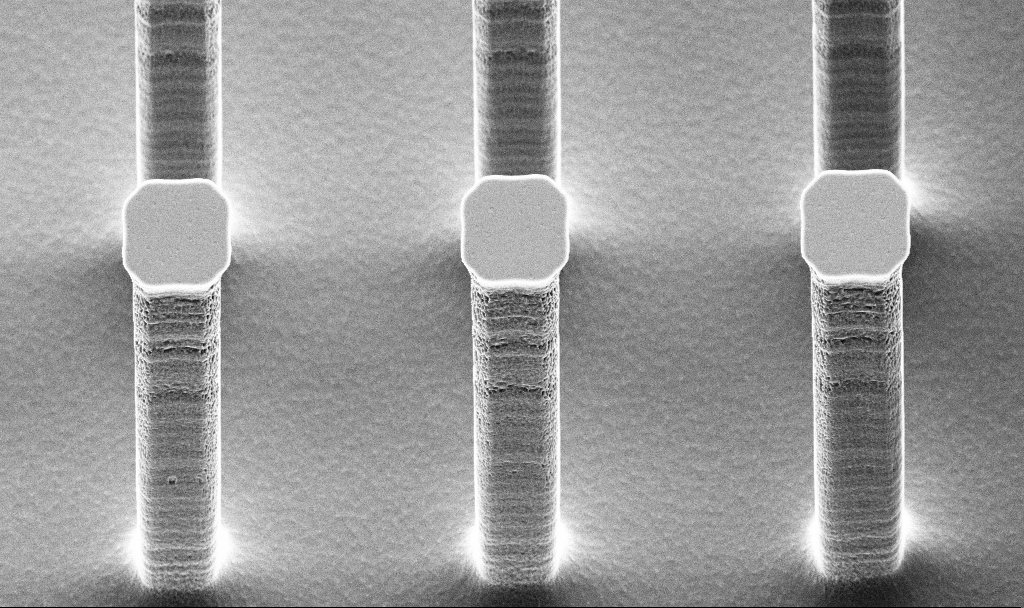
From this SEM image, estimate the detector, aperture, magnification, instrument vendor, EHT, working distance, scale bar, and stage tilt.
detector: SE2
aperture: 30 µm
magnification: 4.14 K X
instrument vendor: Zeiss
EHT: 5 kV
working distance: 13.7 mm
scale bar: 10000 nm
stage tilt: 45°